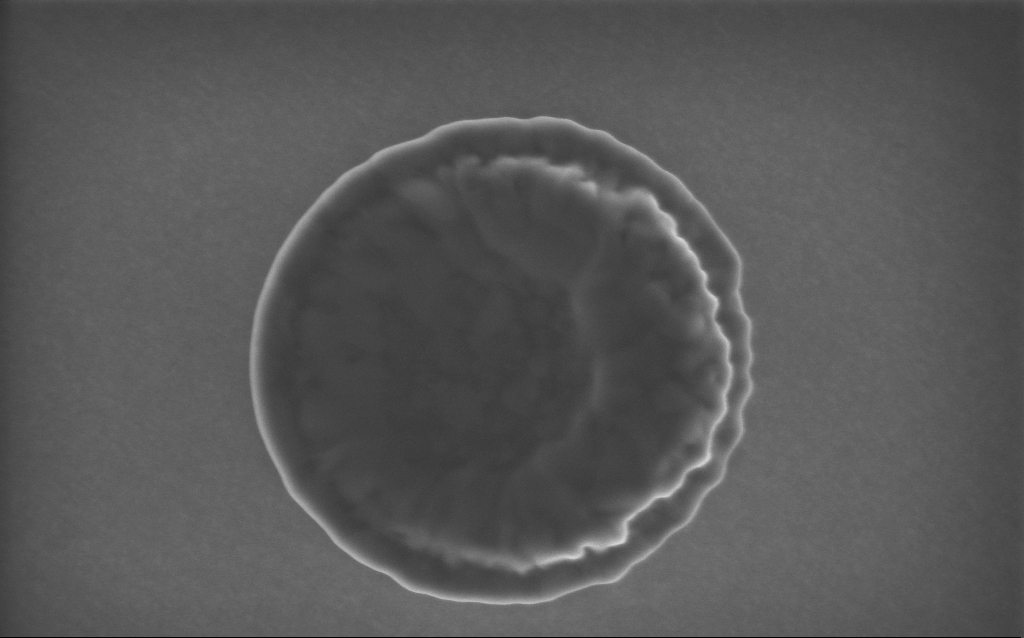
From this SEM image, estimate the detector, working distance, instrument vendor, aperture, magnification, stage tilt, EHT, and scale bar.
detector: InLens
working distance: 4 mm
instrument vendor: Zeiss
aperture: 30 µm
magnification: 82.77 K X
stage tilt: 0°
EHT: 5 kV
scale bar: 200 nm